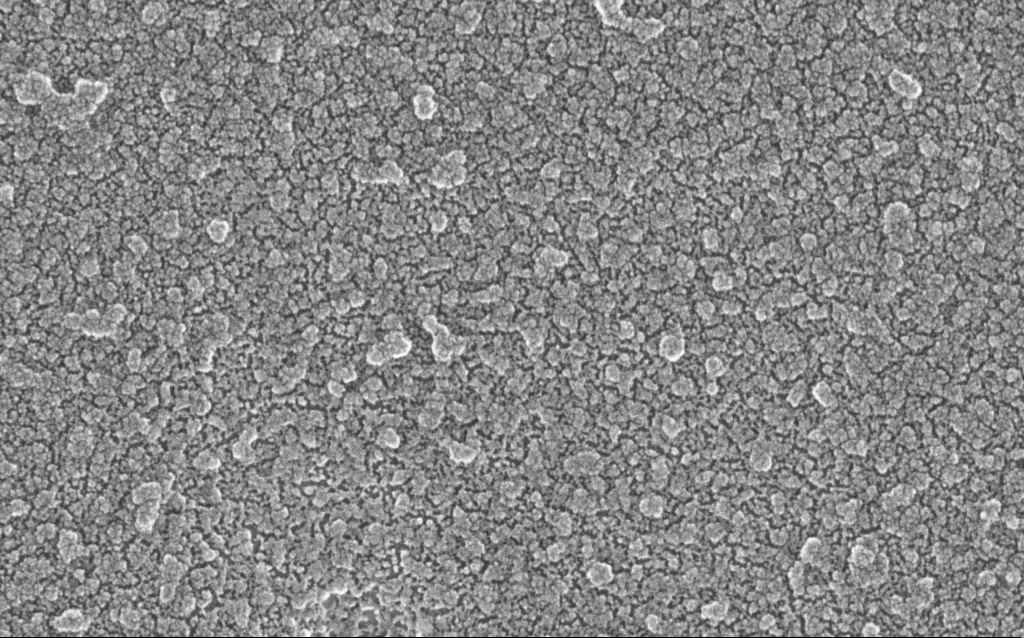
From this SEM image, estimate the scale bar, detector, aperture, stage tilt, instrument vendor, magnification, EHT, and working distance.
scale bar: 100 nm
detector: InLens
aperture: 30 µm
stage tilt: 0°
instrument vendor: Zeiss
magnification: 300 K X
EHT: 20 kV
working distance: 1.6 mm